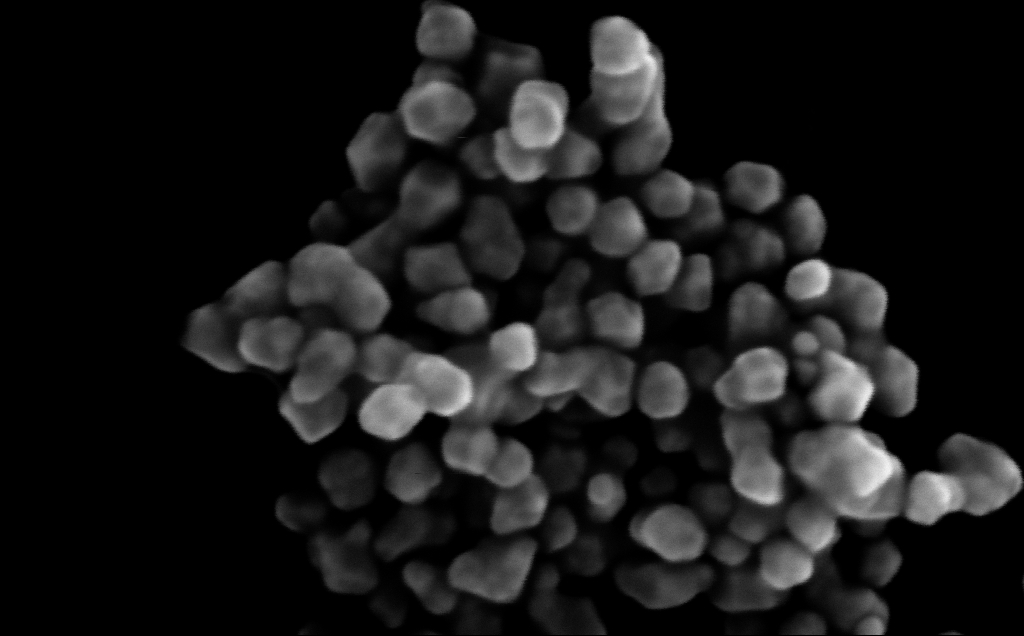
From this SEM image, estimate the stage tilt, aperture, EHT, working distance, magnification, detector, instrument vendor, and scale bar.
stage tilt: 0°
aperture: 30 µm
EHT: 10 kV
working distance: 4 mm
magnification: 524.46 K X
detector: InLens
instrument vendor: Zeiss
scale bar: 100 nm